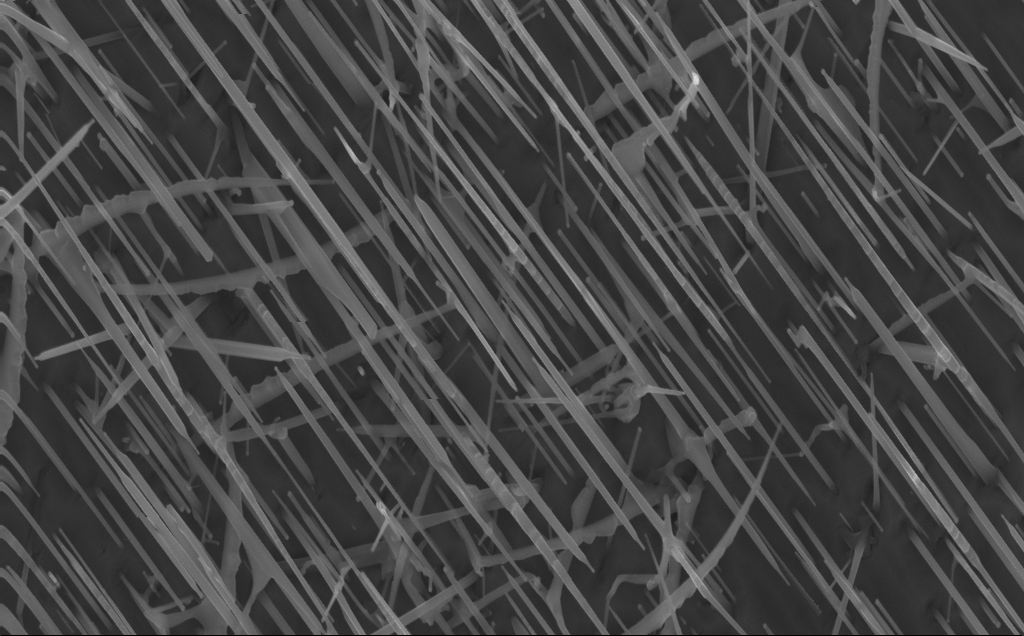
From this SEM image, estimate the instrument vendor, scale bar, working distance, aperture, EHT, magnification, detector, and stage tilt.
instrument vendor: Zeiss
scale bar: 1000 nm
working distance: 4 mm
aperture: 30 µm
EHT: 10 kV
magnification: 40 K X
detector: InLens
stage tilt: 0°